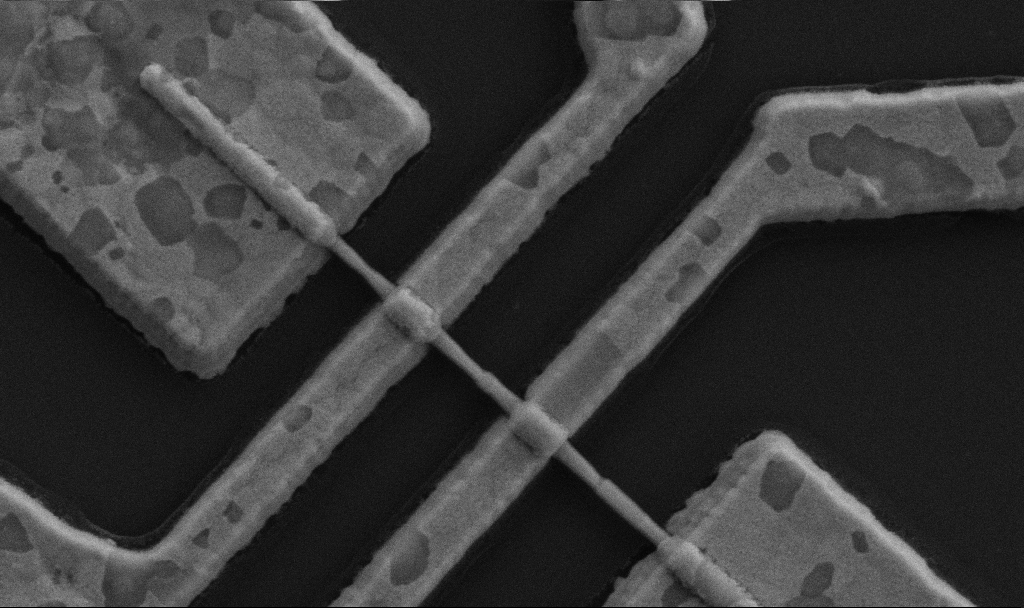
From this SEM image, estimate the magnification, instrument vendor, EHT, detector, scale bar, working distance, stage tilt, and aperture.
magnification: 60 K X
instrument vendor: Zeiss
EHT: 5 kV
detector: SE2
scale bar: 1000 nm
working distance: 10.7 mm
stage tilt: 0°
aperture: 30 µm